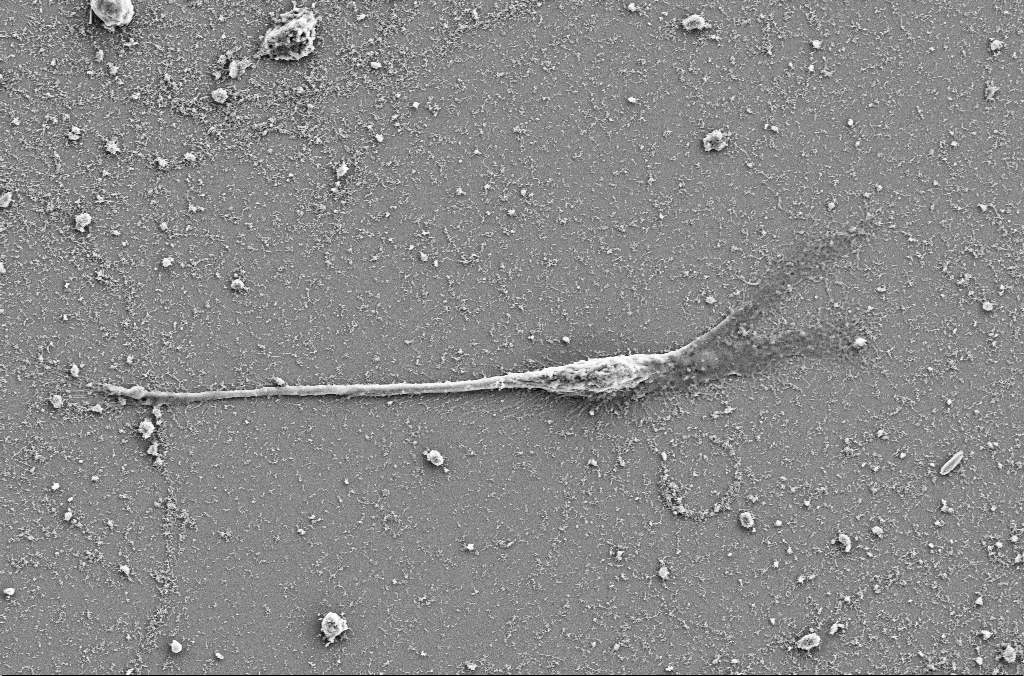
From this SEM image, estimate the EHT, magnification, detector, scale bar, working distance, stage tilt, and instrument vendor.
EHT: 5 kV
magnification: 3.5 K X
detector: SE2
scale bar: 10000 nm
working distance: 4 mm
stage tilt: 0°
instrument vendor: Zeiss